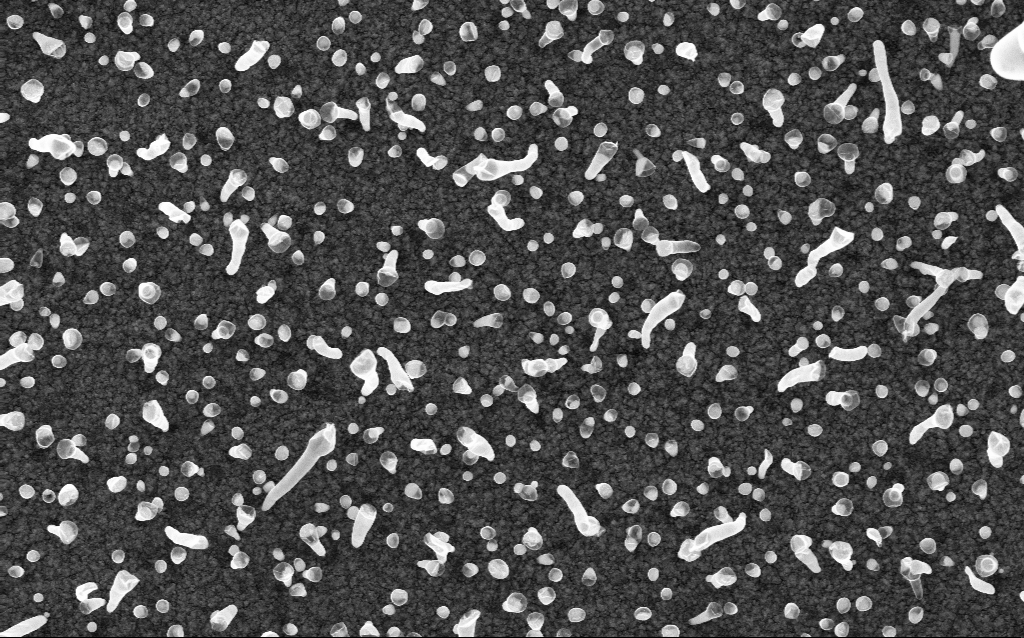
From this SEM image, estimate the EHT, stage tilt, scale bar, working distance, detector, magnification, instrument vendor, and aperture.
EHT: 5 kV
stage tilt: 0°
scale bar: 1000 nm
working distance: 2 mm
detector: InLens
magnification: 50 K X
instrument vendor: Zeiss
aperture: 30 µm